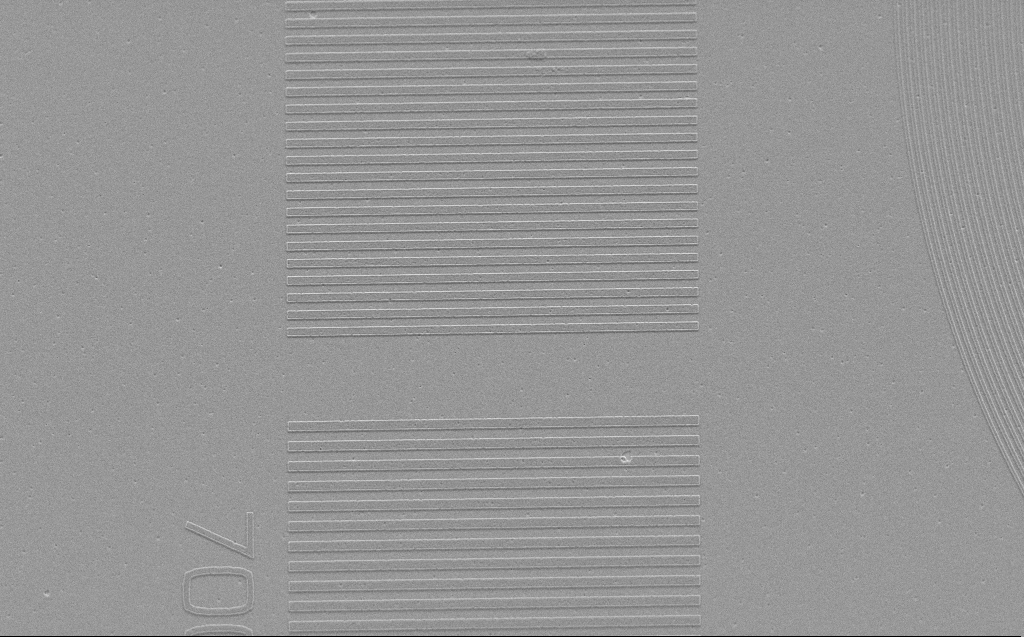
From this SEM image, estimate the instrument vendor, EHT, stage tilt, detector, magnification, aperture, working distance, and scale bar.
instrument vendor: Zeiss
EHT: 3 kV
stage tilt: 41.9°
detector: SE2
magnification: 5.05 K X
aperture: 30 µm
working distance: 9 mm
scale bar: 10000 nm